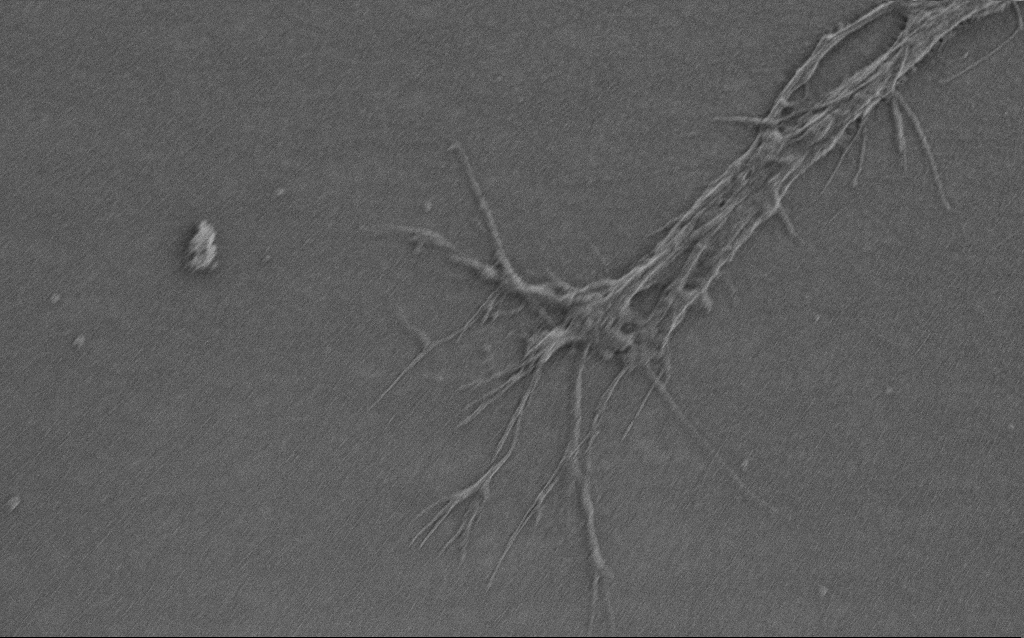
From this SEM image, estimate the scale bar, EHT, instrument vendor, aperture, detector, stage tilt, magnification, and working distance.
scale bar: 2000 nm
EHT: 0.85 kV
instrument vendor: Zeiss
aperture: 30 µm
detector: SE2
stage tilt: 0°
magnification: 7.5 K X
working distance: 6 mm